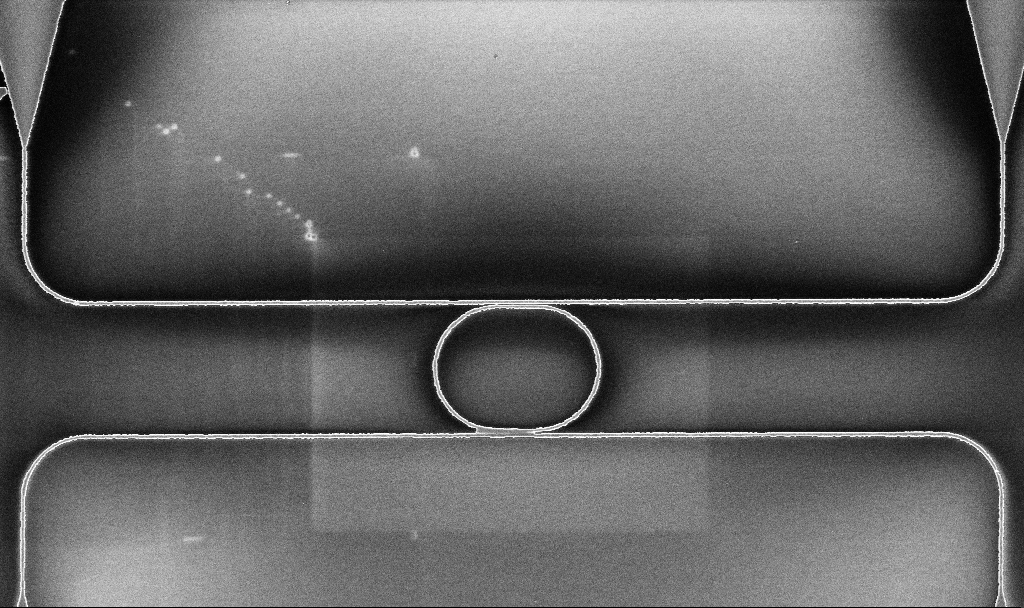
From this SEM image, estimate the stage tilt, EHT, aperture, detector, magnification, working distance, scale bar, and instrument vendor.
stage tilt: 0°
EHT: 5 kV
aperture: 30 µm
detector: InLens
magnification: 2.38 K X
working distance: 10.1 mm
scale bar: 10000 nm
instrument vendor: Zeiss